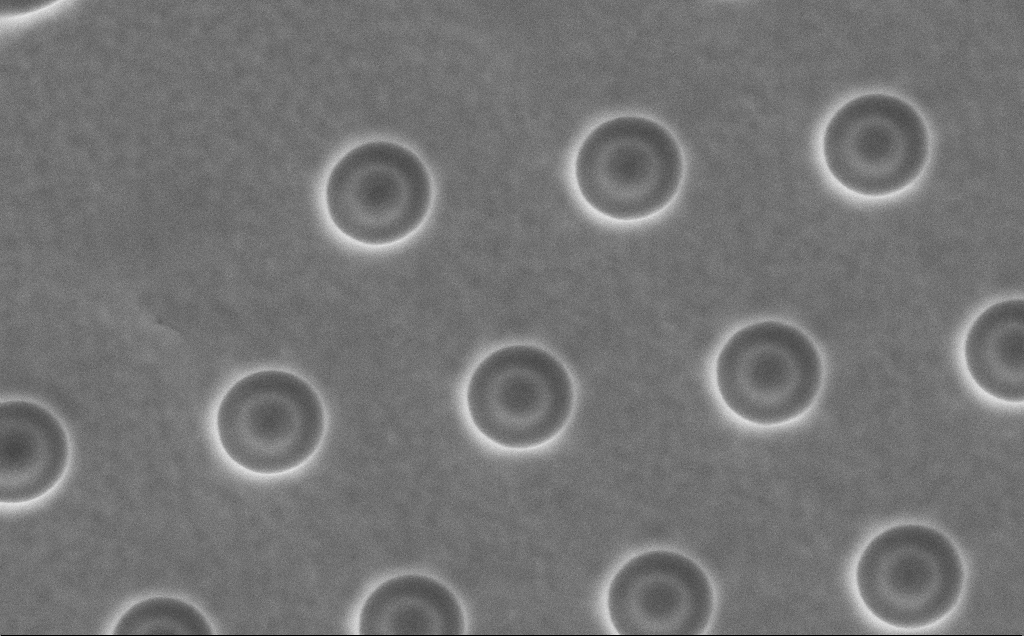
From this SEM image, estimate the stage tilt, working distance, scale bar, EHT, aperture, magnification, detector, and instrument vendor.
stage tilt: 0°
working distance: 11 mm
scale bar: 2000 nm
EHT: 5 kV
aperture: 30 µm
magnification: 14.34 K X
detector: SE2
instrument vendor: Zeiss